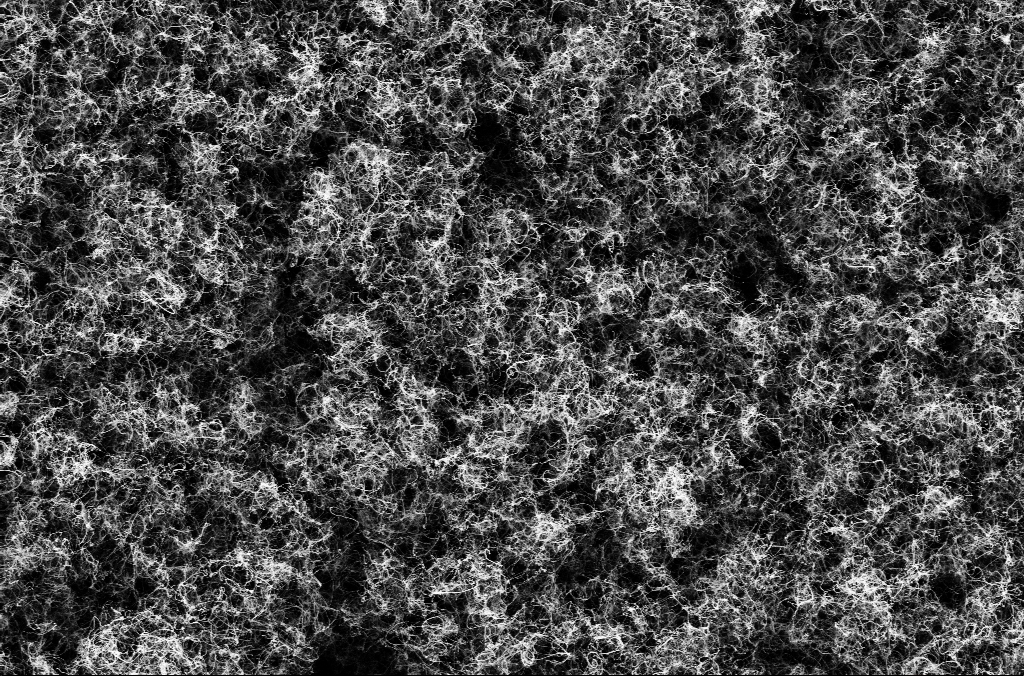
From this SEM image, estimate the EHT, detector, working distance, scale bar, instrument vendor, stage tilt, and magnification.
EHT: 1.8 kV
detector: SE2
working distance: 5.3 mm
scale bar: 2000 nm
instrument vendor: Zeiss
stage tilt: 0°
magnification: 10 K X